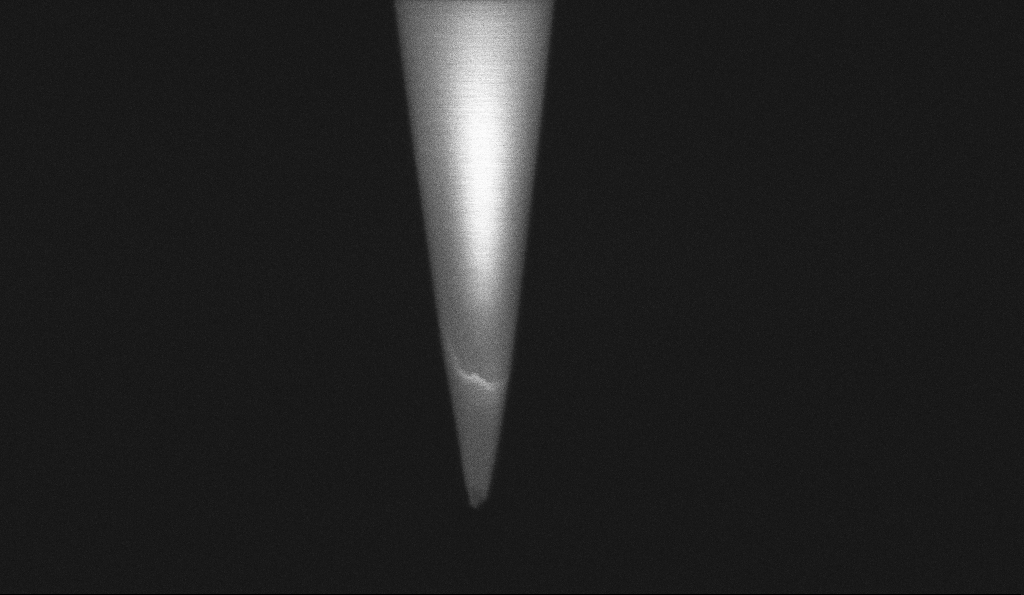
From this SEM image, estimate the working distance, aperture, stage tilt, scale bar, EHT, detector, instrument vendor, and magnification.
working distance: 5.6 mm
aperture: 30 µm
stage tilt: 0°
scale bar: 200 nm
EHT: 1 kV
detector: InLens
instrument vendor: Zeiss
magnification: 100 K X